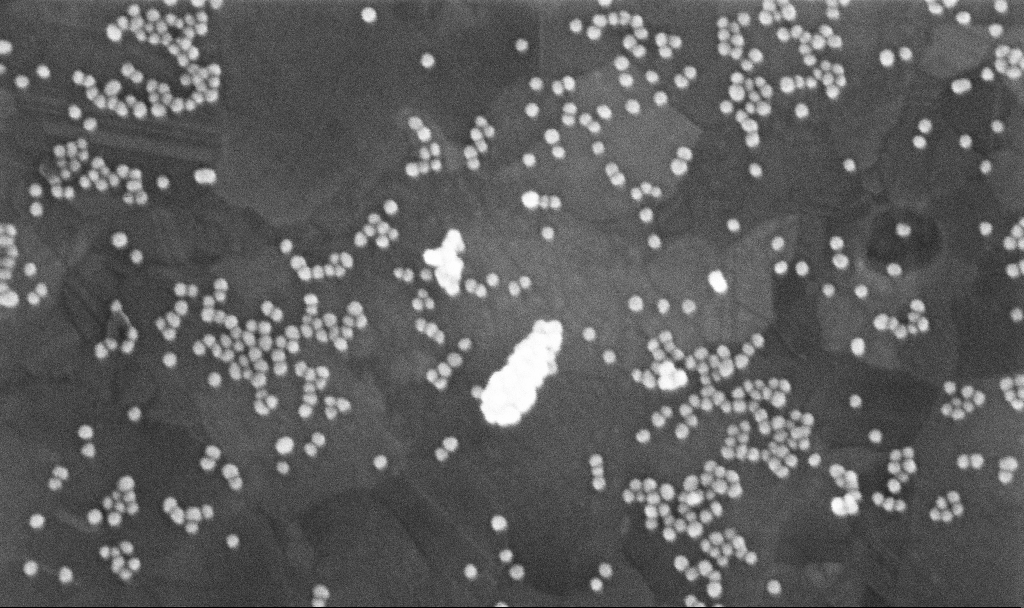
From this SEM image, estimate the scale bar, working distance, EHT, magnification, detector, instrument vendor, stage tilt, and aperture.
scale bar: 200 nm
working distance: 3.7 mm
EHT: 10 kV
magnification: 266.66 K X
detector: InLens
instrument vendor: Zeiss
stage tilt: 0°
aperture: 30 µm